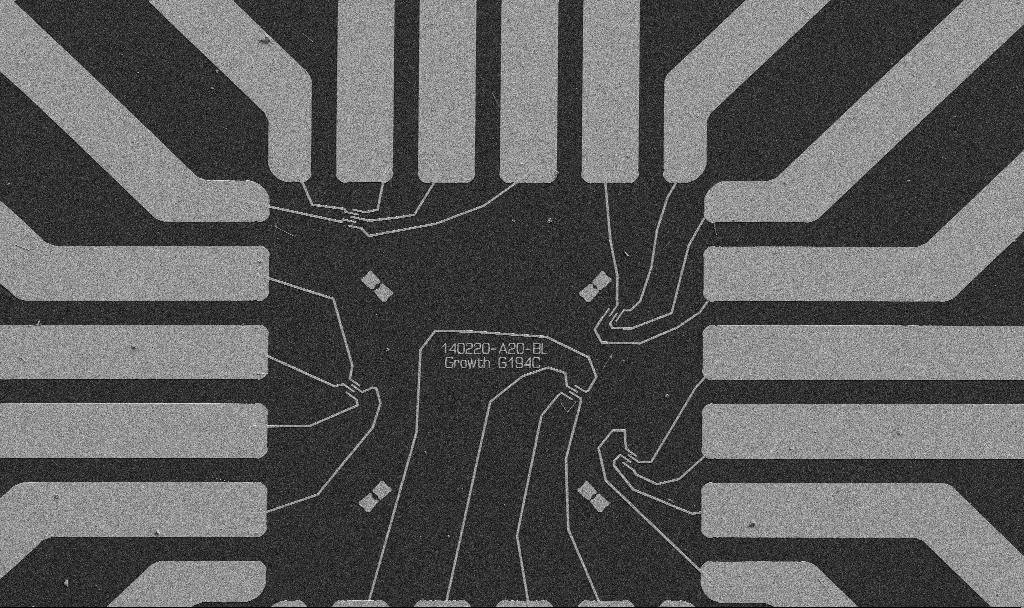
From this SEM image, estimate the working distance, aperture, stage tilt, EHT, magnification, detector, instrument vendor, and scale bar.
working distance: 10.7 mm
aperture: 30 µm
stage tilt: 0°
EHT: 5 kV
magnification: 1 K X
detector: SE2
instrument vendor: Zeiss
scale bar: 20000 nm